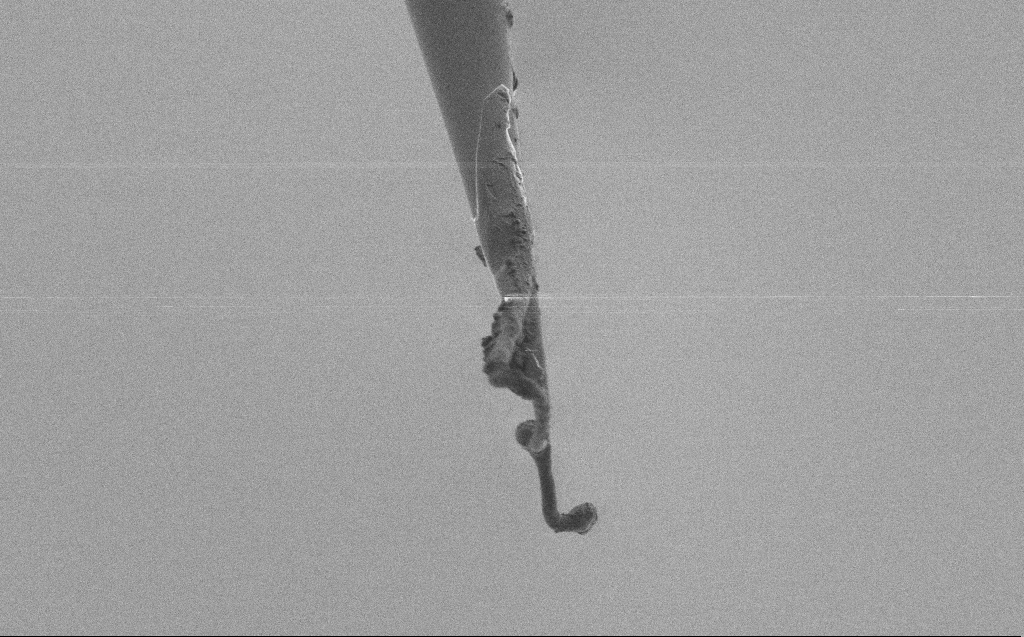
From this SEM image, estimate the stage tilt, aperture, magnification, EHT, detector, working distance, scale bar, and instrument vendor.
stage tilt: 39.3°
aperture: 10 µm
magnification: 5 K X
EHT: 0.5 kV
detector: SE2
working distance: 5 mm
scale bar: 10000 nm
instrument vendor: Zeiss